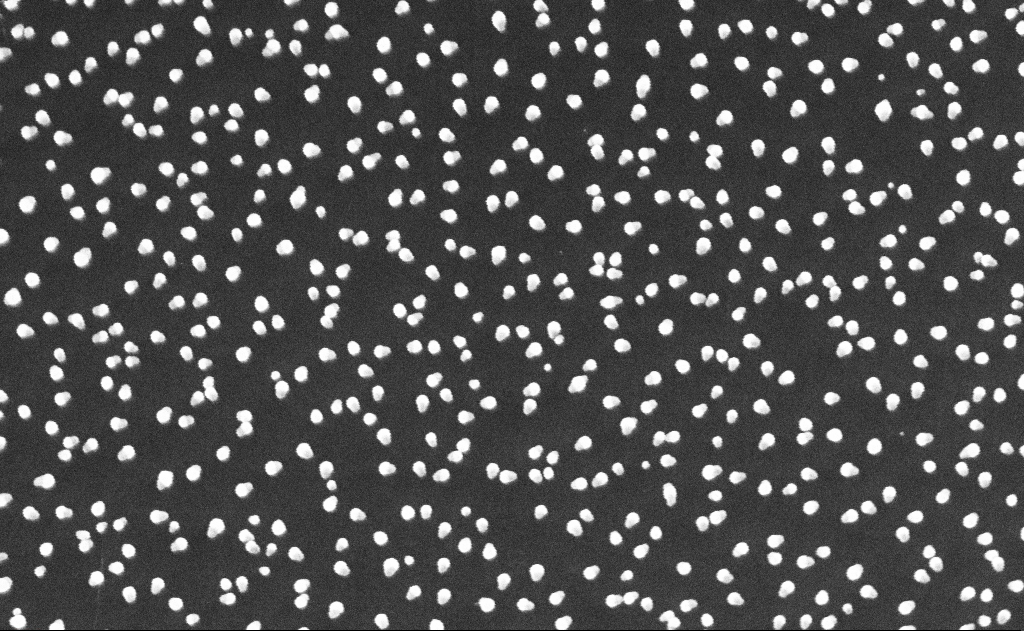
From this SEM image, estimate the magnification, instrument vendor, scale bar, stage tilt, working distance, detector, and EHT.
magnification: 100 K X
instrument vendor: Zeiss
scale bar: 200 nm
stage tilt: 0°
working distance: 12 mm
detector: InLens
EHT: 10 kV